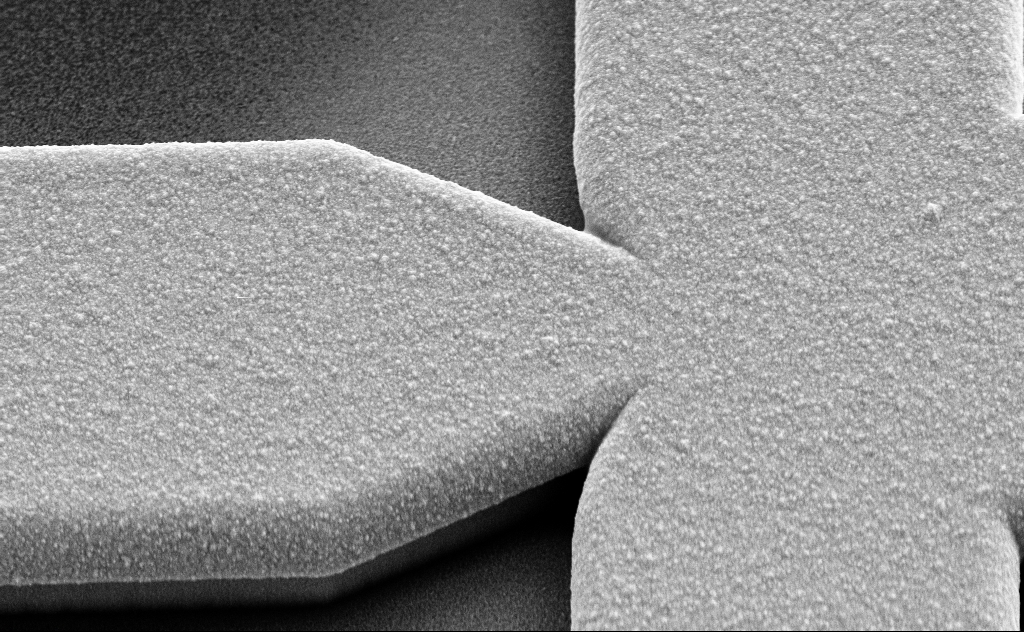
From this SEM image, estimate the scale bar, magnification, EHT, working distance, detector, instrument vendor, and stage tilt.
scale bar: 2000 nm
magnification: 13.17 K X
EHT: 10 kV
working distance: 11 mm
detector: SE2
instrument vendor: Zeiss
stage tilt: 45°